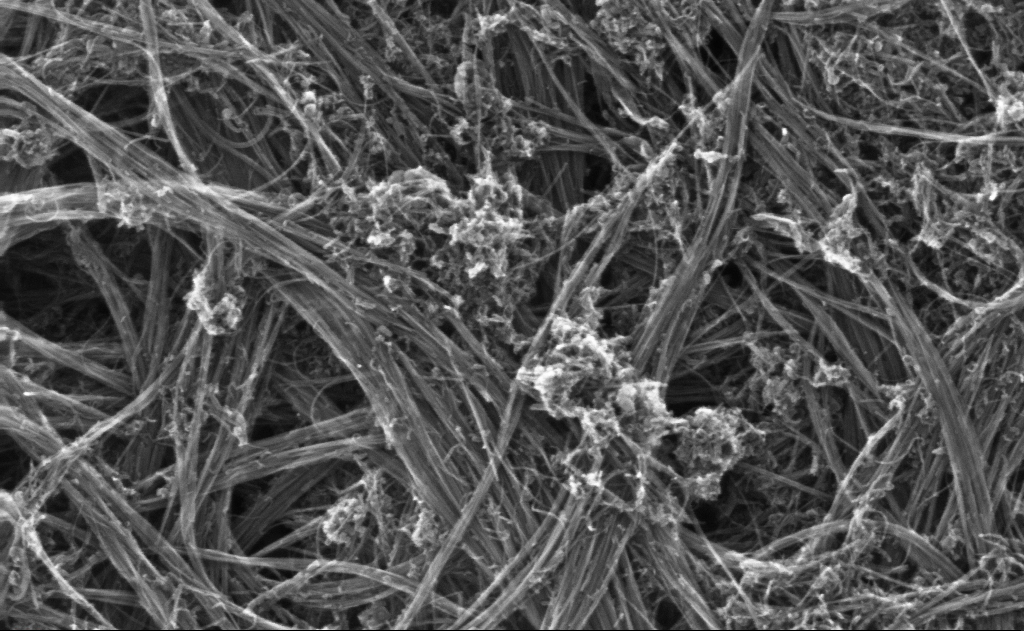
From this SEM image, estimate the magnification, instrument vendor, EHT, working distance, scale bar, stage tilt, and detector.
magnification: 233.12 K X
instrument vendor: Zeiss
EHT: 10 kV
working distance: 3 mm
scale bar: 200 nm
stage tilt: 0°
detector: InLens